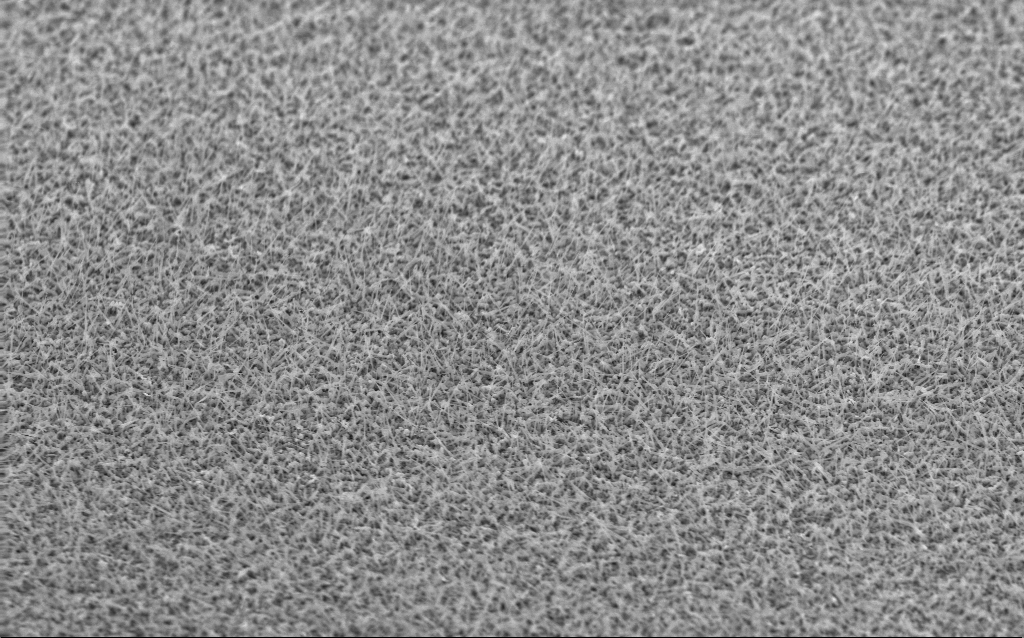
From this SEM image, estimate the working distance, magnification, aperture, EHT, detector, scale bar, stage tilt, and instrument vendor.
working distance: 3 mm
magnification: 10 K X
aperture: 30 µm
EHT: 10 kV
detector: InLens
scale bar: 2000 nm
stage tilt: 53°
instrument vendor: Zeiss